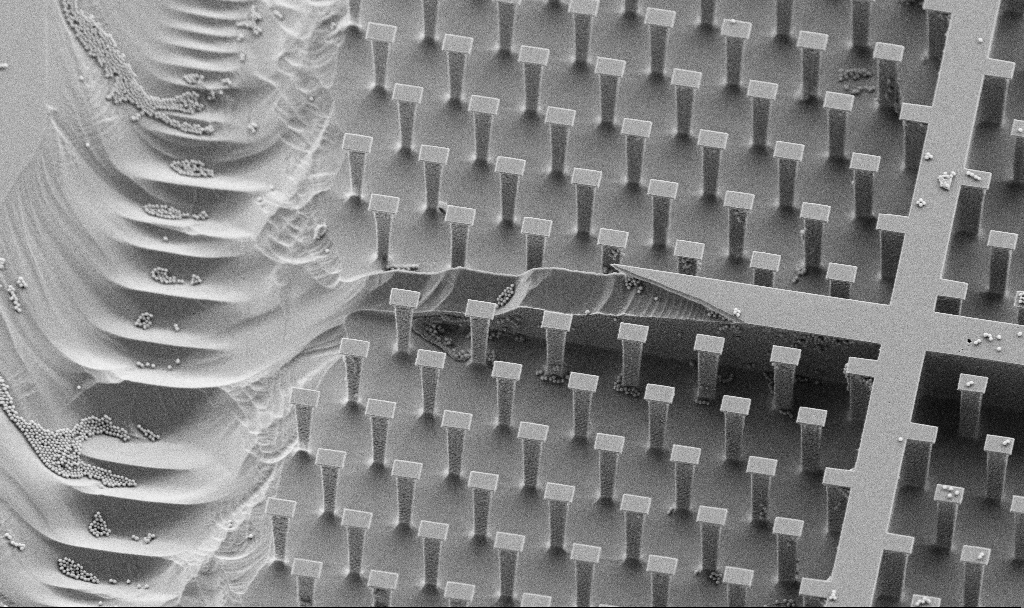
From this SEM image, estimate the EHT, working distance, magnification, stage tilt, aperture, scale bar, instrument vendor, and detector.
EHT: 5 kV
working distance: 4.5 mm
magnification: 2.37 K X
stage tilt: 30°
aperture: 30 µm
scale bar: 10000 nm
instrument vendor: Zeiss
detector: SE2